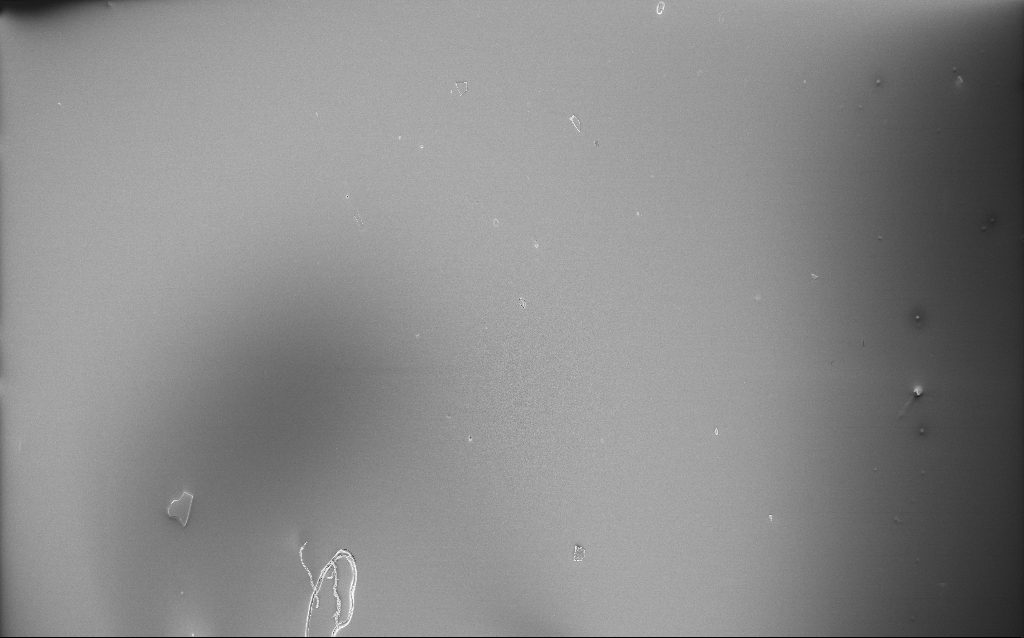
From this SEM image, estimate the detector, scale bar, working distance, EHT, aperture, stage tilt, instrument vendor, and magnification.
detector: InLens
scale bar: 200000 nm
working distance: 2.6 mm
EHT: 5 kV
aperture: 30 µm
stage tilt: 0°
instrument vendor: Zeiss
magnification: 0.11 K X